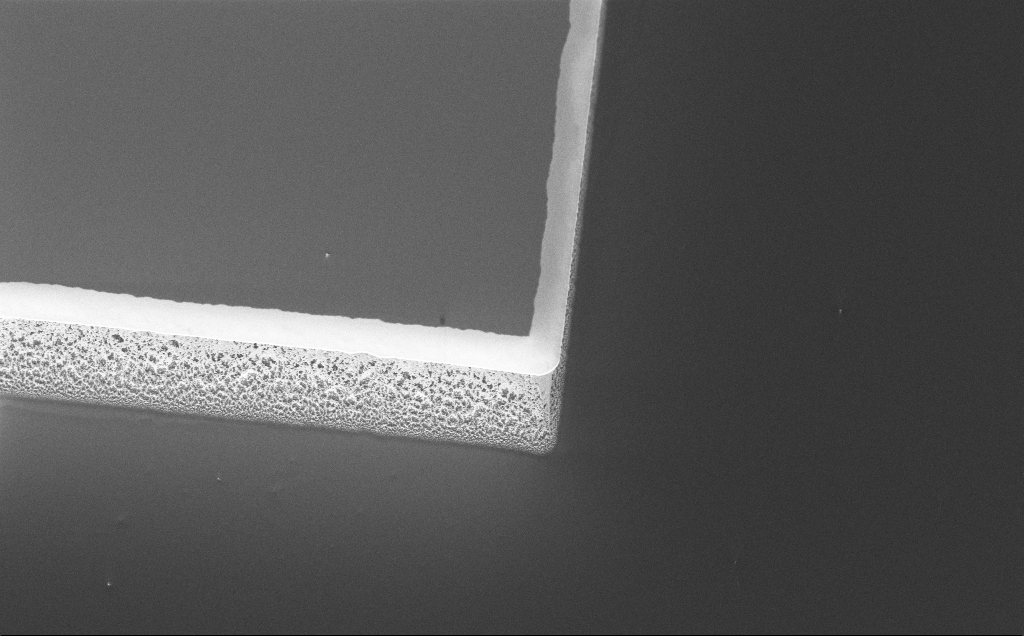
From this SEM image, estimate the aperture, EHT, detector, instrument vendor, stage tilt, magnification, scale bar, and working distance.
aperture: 30 µm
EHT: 5 kV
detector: InLens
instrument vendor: Zeiss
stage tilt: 45°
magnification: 2.45 K X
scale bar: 20000 nm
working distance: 8 mm